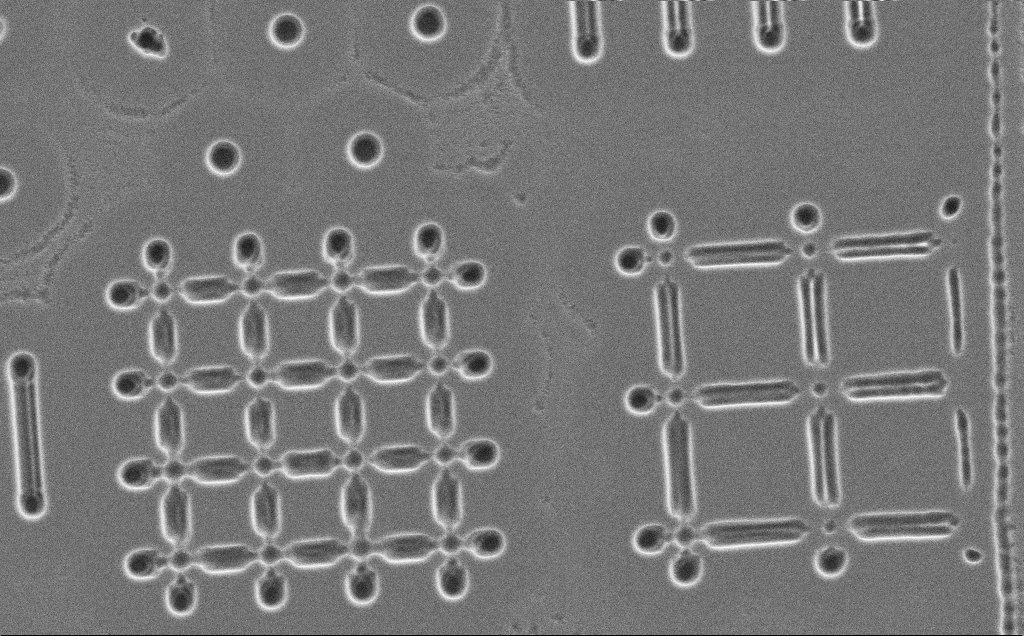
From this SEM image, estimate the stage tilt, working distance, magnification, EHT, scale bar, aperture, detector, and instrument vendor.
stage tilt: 0°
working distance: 13 mm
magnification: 5.15 K X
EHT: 5 kV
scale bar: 10000 nm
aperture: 30 µm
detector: SE2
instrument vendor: Zeiss